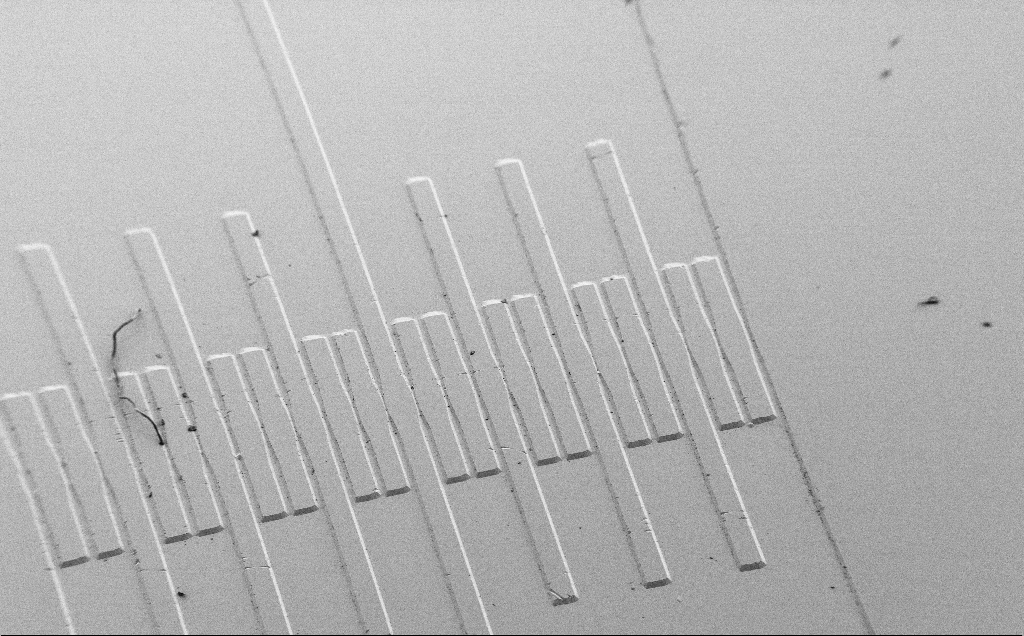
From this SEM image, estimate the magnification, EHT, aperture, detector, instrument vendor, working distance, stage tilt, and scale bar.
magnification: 0.107 K X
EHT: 3 kV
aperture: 30 µm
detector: SE2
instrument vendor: Zeiss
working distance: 9 mm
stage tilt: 45°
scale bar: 200000 nm